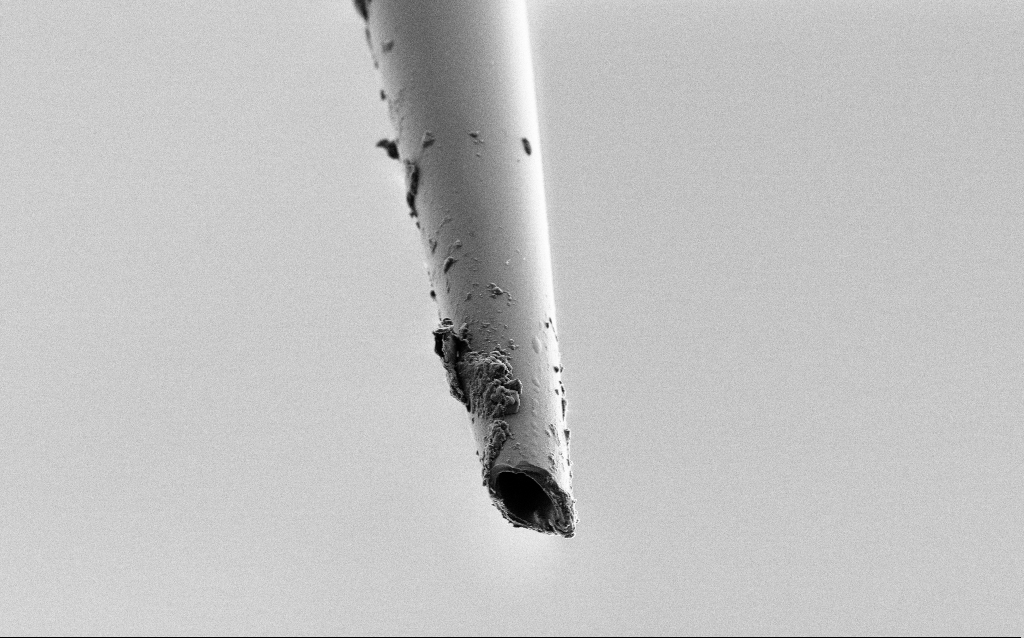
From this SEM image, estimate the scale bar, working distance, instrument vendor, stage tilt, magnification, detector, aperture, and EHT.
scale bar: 10000 nm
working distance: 6 mm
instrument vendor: Zeiss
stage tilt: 45°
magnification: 5 K X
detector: SE2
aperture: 30 µm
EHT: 2 kV